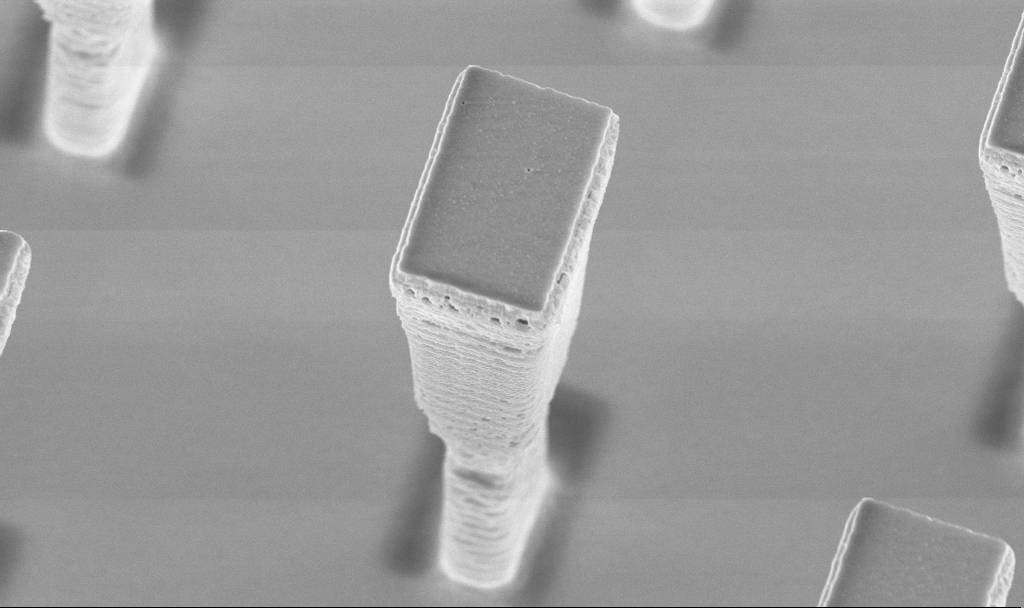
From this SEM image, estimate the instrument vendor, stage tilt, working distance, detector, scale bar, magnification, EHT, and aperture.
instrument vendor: Zeiss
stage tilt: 20°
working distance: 4.1 mm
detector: InLens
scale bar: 2000 nm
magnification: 19.01 K X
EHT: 5 kV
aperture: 30 µm